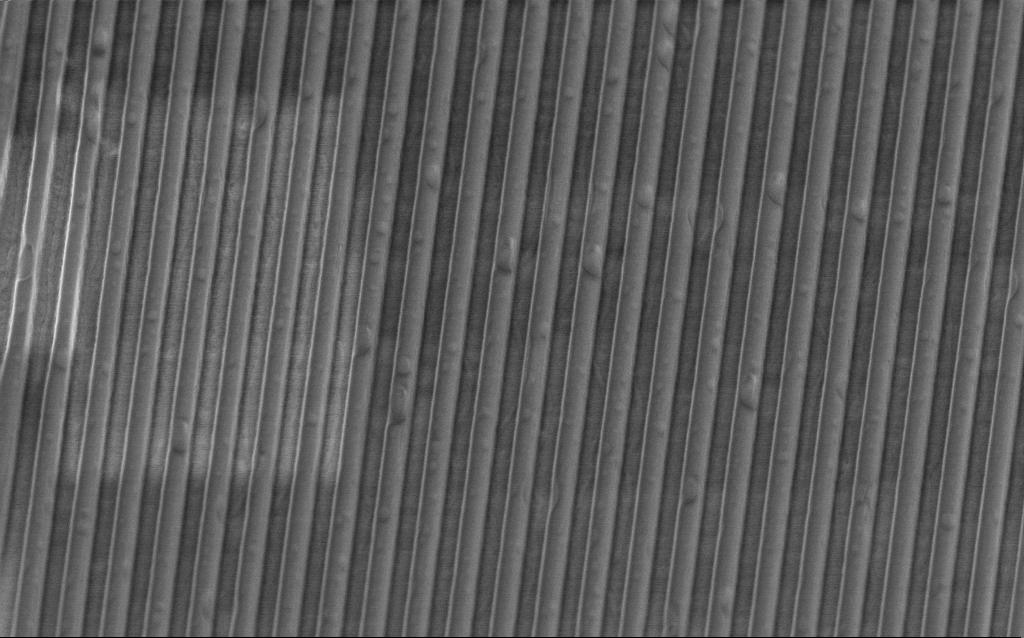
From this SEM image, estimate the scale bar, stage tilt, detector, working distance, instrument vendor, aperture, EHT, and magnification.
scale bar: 1000 nm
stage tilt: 45°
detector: InLens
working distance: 3.8 mm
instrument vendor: Zeiss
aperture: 30 µm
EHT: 2 kV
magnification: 31.58 K X